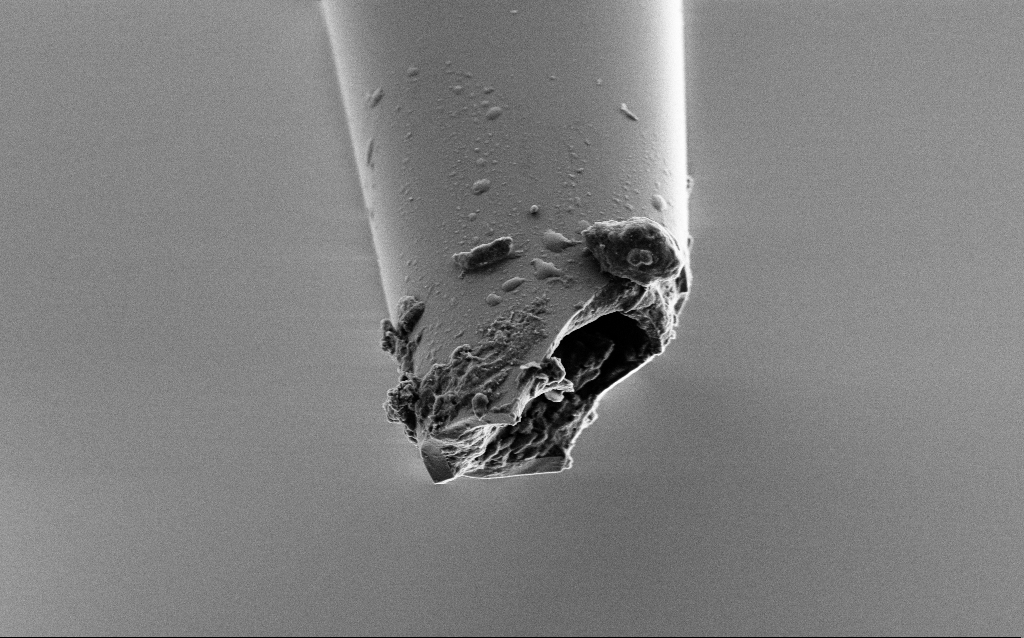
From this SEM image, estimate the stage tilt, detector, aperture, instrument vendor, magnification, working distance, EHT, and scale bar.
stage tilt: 45°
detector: SE2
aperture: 30 µm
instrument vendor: Zeiss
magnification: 10 K X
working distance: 6 mm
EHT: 2 kV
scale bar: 2000 nm